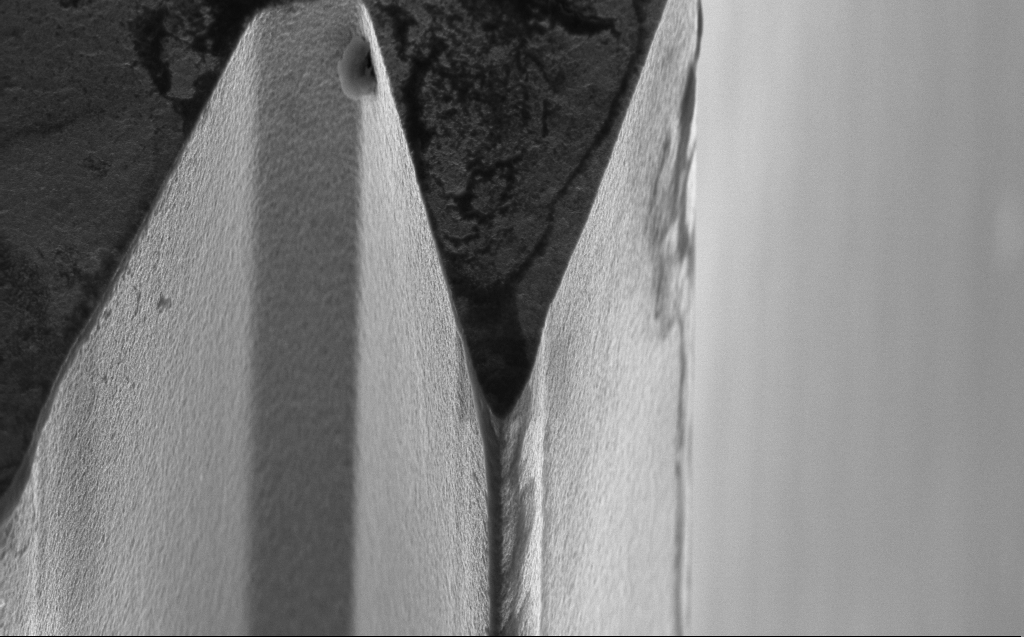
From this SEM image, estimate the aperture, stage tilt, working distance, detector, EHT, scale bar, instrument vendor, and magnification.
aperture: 30 µm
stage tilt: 45°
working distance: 5 mm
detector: InLens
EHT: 5 kV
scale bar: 1000 nm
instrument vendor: Zeiss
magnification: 17.62 K X